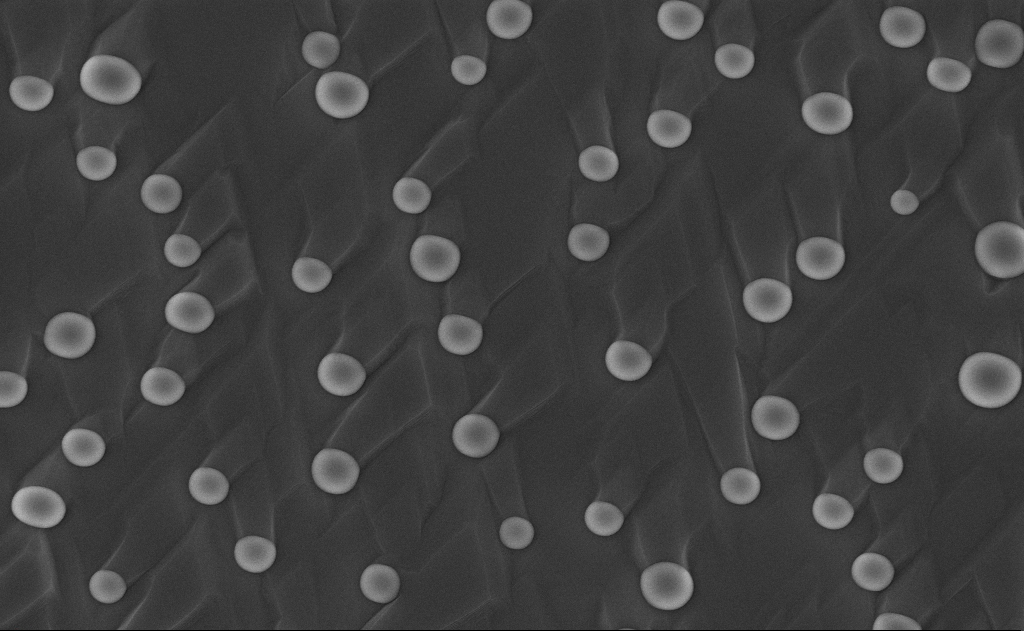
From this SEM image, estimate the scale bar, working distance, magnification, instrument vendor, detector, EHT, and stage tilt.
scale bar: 2000 nm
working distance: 10 mm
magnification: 20 K X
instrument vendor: Zeiss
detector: InLens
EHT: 10 kV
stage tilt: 0°